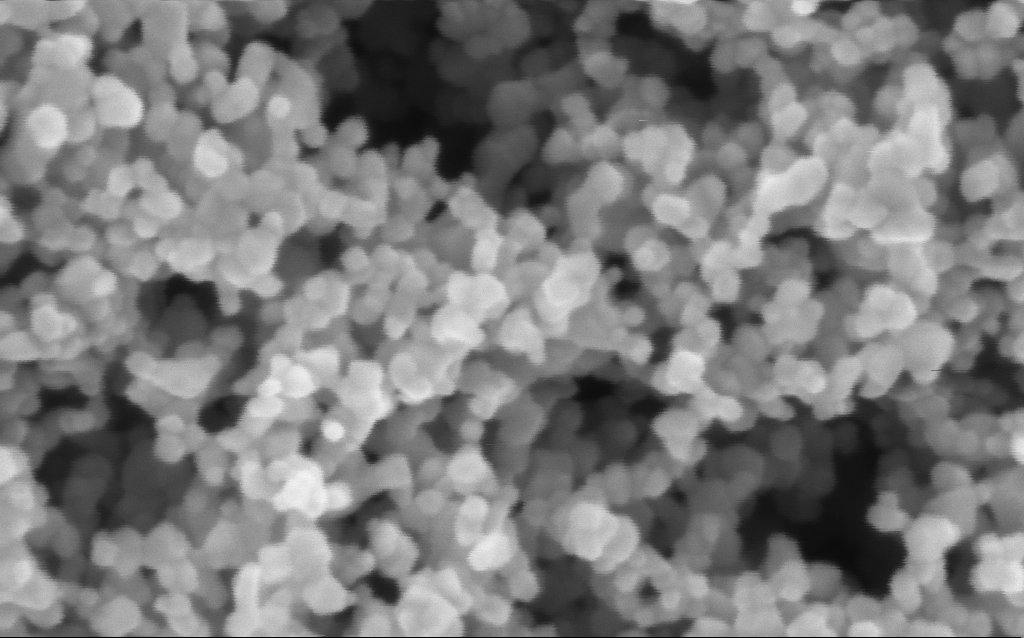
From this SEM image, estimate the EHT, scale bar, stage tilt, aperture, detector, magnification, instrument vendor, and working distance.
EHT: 3 kV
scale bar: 100 nm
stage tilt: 0°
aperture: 30 µm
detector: InLens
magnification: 416 K X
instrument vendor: Zeiss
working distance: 7.5 mm